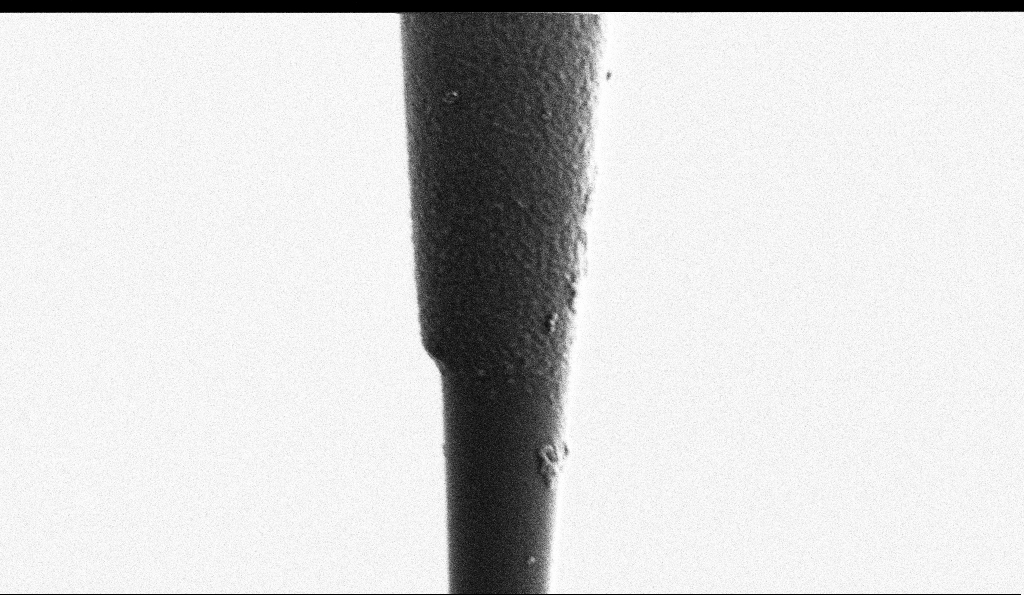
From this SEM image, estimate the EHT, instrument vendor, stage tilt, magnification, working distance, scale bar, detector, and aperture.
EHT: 1 kV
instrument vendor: Zeiss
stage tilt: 0°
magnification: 25 K X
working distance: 6.5 mm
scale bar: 2000 nm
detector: SE2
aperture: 30 µm